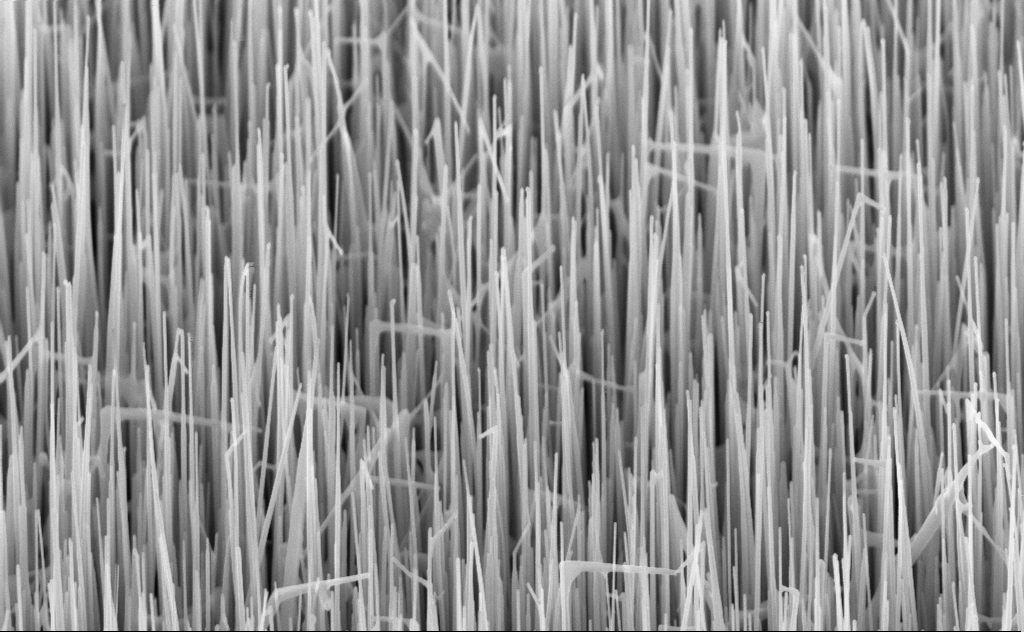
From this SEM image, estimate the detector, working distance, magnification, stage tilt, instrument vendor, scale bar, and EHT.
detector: InLens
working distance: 6 mm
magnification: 40 K X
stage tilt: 45°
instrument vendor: Zeiss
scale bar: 1000 nm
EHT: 10 kV